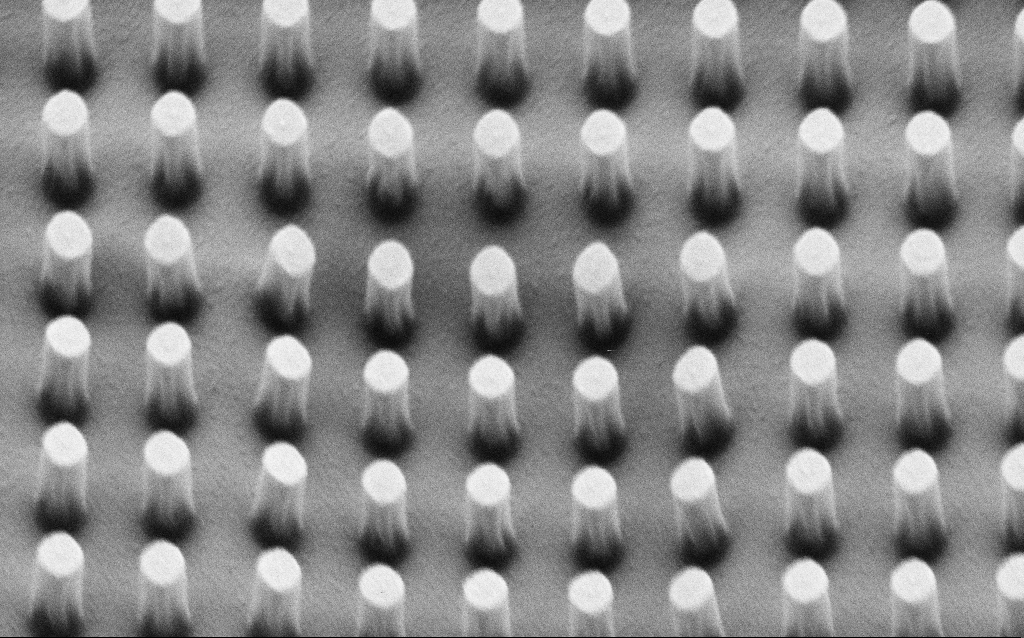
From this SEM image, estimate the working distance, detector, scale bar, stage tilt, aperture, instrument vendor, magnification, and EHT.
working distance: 4.7 mm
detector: SE2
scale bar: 200 nm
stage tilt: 45°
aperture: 30 µm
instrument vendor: Zeiss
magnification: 80.23 K X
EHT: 3 kV